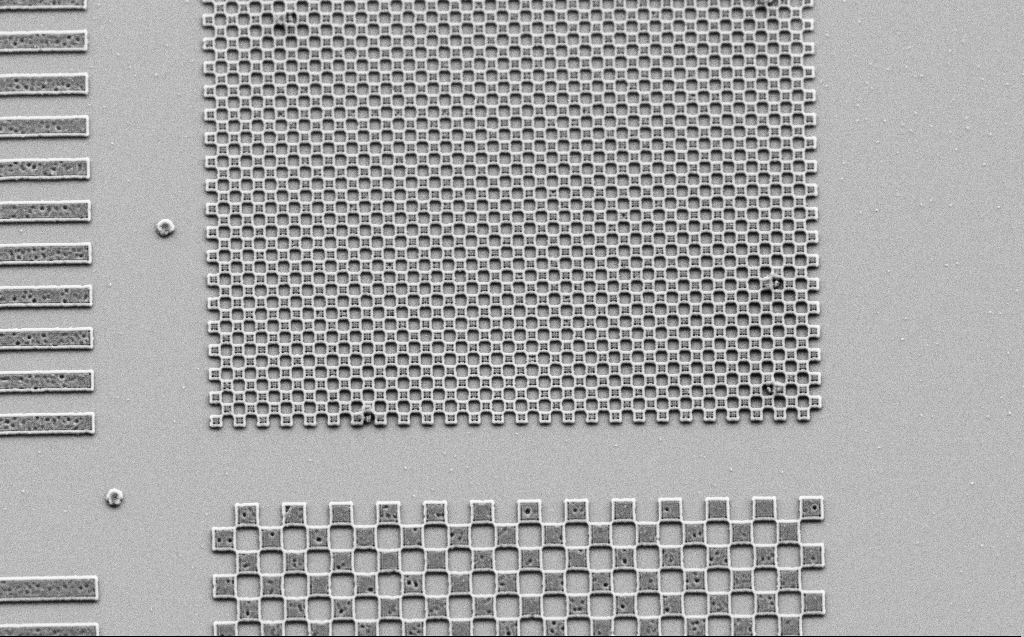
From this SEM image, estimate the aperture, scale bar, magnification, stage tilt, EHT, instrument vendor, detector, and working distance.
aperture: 30 µm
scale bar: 2000 nm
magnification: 8.72 K X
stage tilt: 30°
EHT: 3 kV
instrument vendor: Zeiss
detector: SE2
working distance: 6 mm